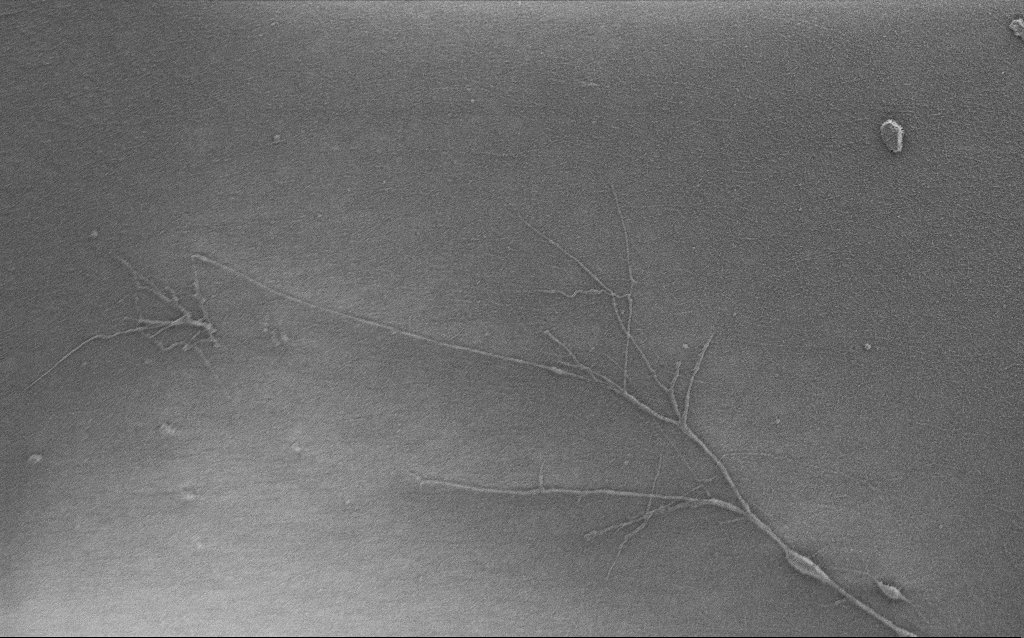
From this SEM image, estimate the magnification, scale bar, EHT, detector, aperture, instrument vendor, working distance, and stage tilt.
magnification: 5 K X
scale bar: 10000 nm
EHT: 0.9 kV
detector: SE2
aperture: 30 µm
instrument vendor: Zeiss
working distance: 6 mm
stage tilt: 0°